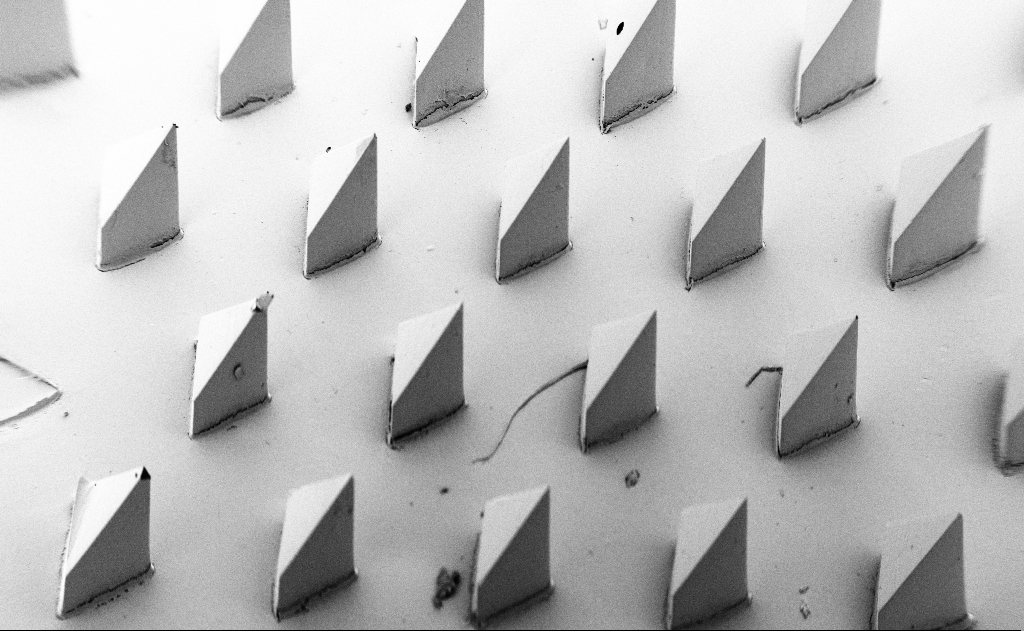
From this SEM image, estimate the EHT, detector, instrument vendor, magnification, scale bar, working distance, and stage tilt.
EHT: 5 kV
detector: SE2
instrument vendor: Zeiss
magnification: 0.071 K X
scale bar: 1e+06 nm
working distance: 8 mm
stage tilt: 45°